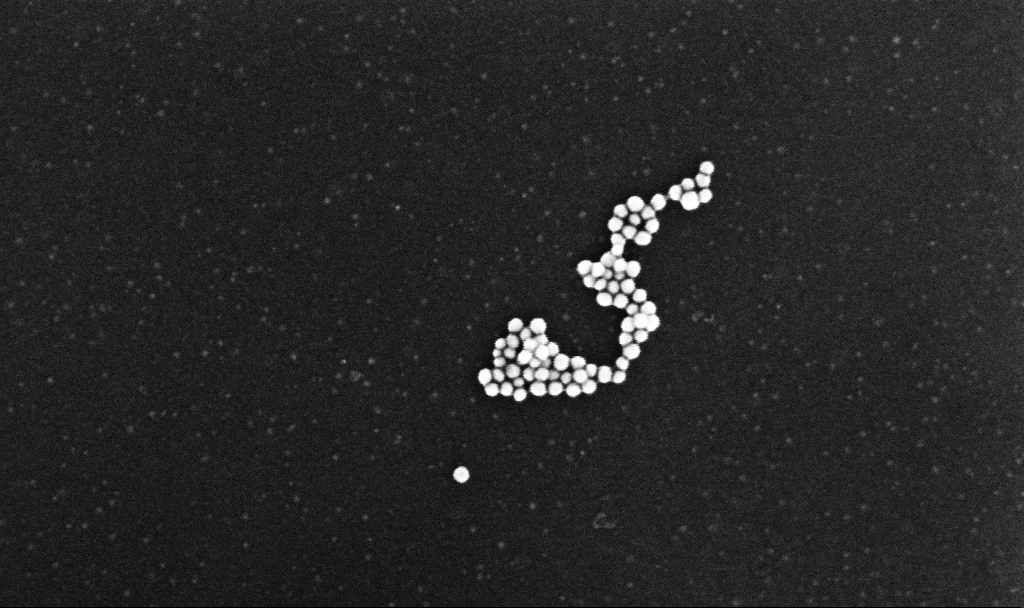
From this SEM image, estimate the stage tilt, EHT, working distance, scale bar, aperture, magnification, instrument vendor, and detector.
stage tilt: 0°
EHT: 10 kV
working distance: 3.2 mm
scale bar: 100 nm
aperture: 30 µm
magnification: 236.2 K X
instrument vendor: Zeiss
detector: InLens